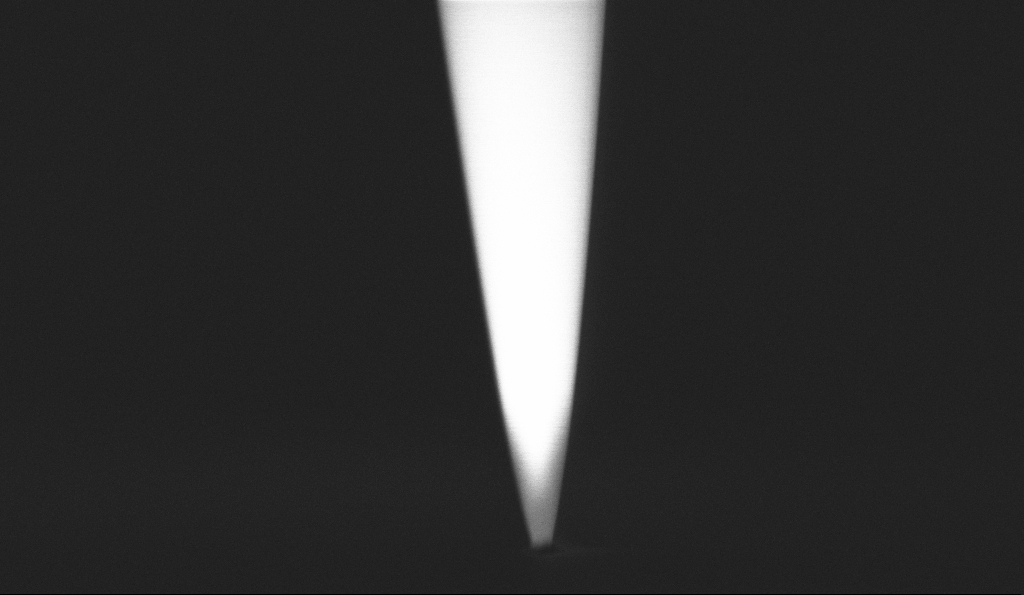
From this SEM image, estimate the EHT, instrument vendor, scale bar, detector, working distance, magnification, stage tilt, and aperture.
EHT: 1 kV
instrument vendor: Zeiss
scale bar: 1000 nm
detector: InLens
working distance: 5.7 mm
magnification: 50 K X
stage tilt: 0°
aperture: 30 µm